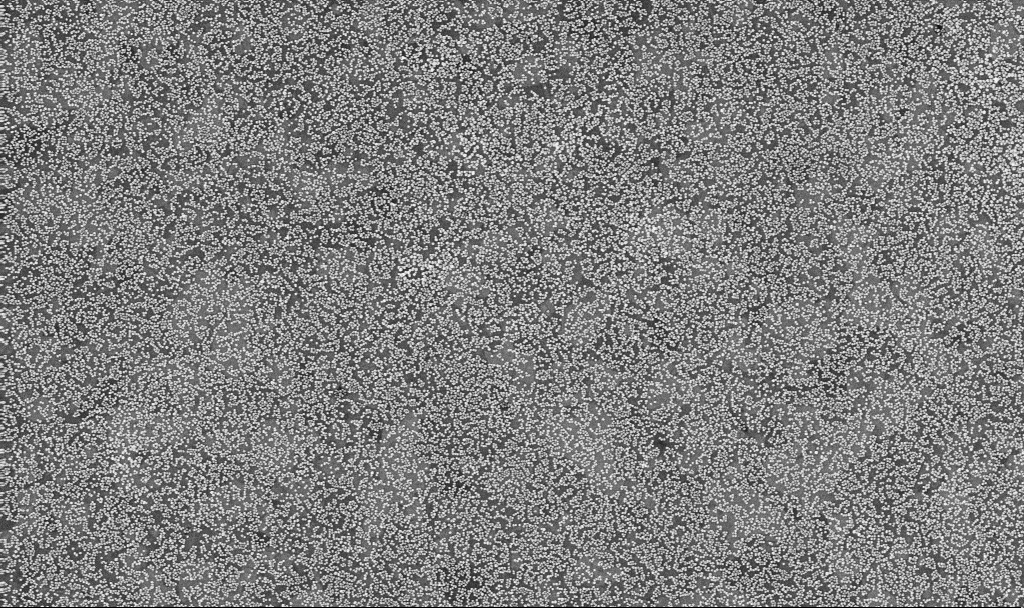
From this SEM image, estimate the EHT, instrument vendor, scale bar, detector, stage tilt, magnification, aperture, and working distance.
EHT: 10 kV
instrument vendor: Zeiss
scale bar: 1000 nm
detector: InLens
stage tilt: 0°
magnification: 50 K X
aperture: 30 µm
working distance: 3.3 mm